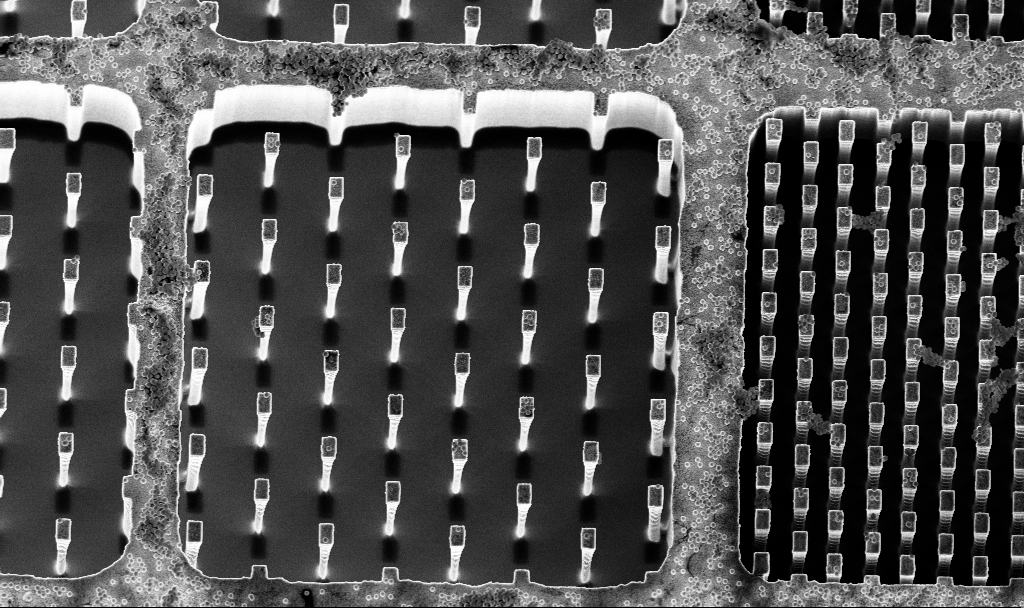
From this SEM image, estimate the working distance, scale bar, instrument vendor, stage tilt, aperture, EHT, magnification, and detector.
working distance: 3.2 mm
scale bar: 10000 nm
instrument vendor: Zeiss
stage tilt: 15°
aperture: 30 µm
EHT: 5 kV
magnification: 3.39 K X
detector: InLens